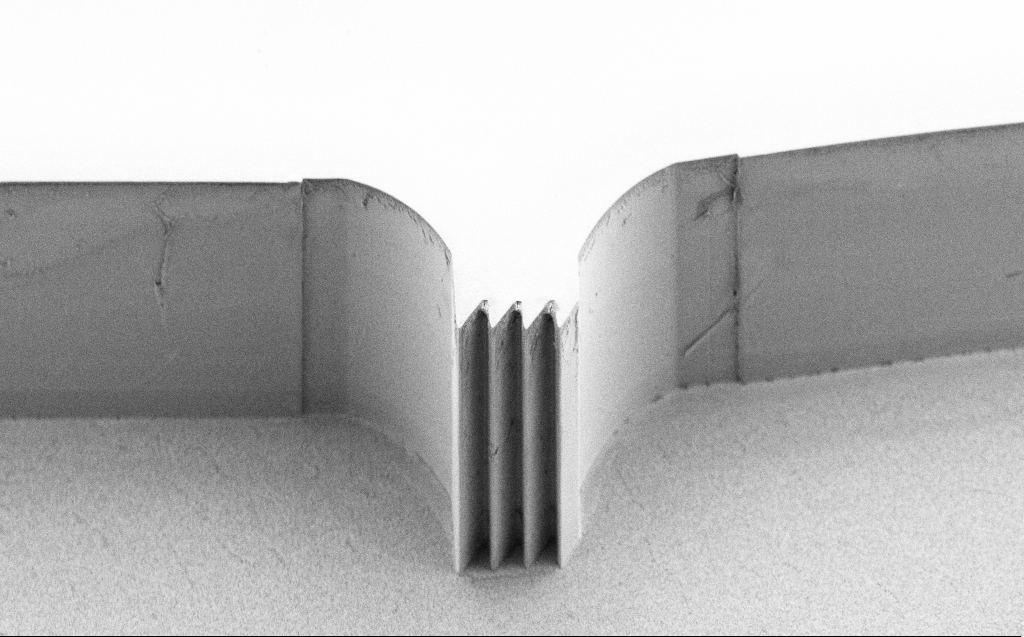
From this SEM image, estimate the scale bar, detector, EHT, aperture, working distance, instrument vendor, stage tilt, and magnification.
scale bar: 20000 nm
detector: SE2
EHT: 5 kV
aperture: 30 µm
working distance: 7 mm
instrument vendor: Zeiss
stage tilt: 45°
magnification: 1.08 K X